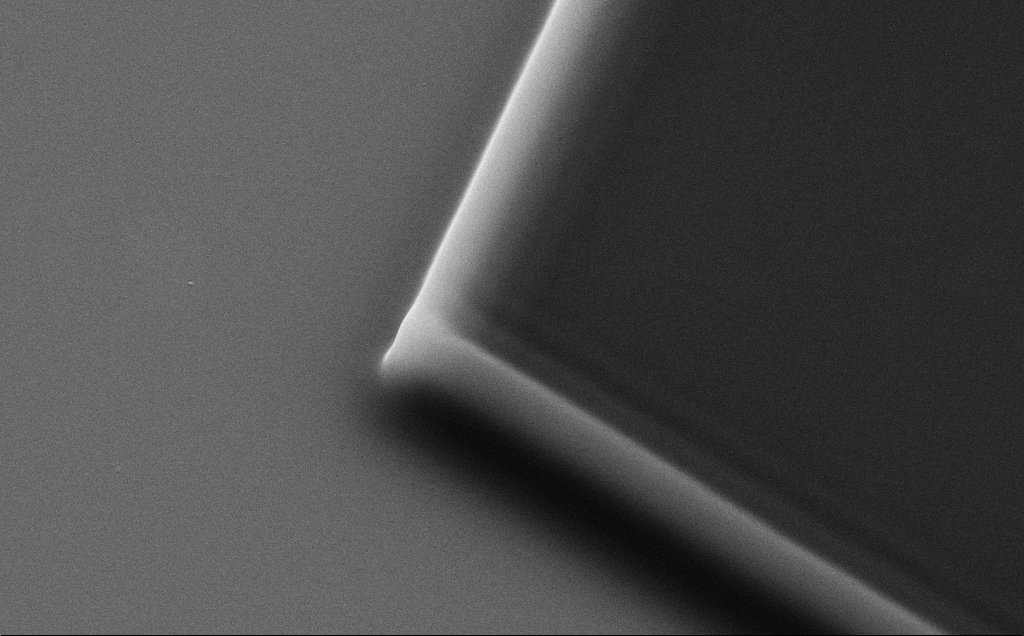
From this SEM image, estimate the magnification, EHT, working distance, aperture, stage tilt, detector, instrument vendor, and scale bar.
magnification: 25.56 K X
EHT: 10 kV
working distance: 8 mm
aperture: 30 µm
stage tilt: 35°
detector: SE2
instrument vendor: Zeiss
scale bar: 1000 nm